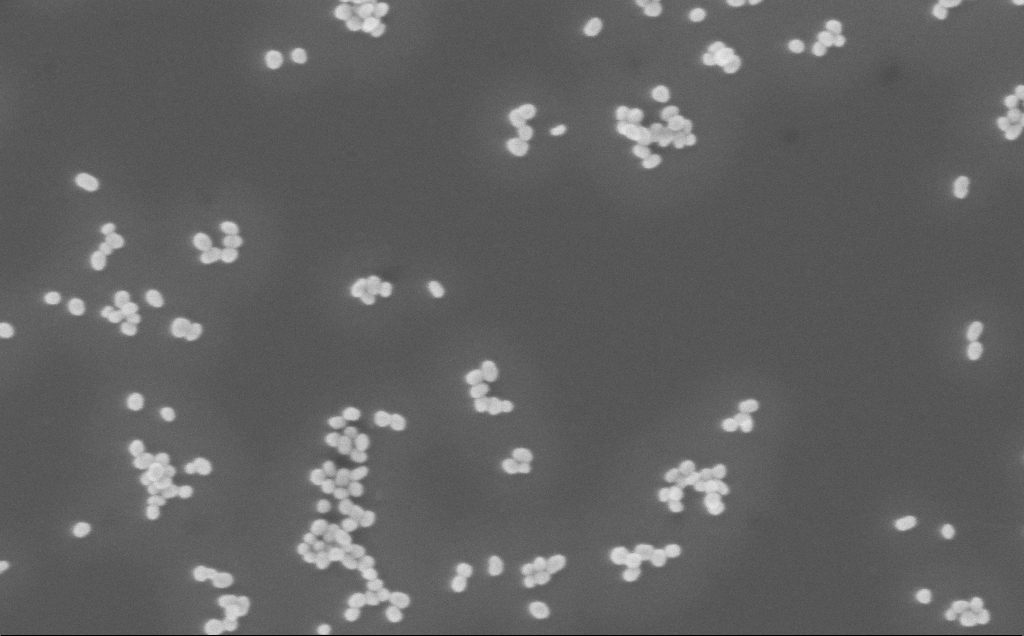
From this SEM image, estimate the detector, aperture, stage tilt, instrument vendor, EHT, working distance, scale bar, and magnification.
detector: InLens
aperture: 30 µm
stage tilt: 0°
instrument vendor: Zeiss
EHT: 3 kV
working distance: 5 mm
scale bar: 100 nm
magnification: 150 K X